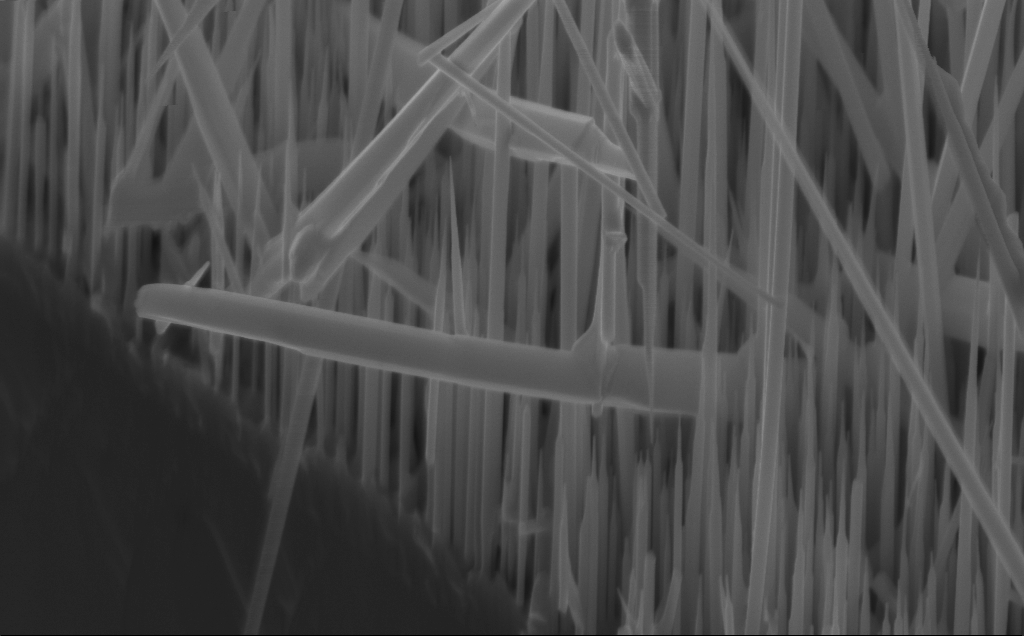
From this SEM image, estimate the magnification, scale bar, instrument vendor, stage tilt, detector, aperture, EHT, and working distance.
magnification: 50.76 K X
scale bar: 1000 nm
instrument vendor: Zeiss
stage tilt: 45°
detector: InLens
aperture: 30 µm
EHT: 10 kV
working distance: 5 mm